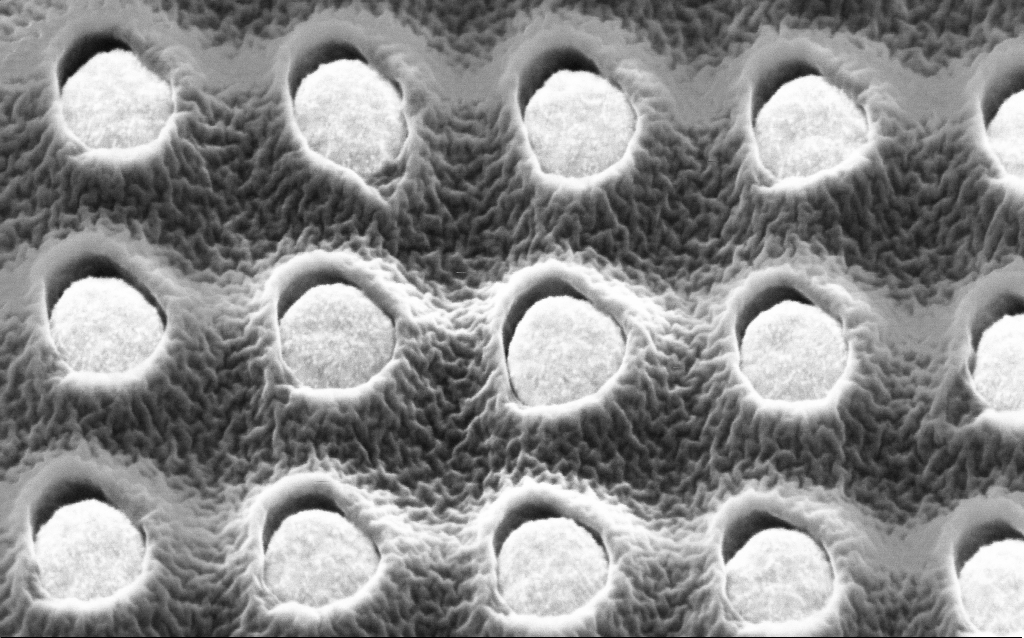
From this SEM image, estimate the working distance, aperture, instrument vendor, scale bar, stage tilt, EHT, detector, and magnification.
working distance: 3.5 mm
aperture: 30 µm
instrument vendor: Zeiss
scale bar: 200 nm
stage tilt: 45°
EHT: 2 kV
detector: InLens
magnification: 171.08 K X